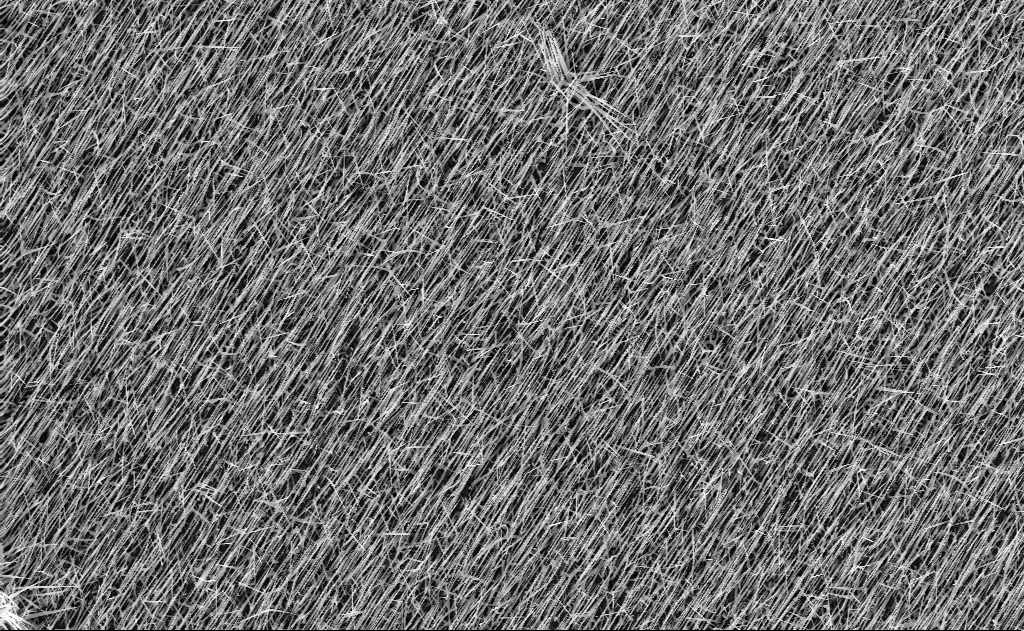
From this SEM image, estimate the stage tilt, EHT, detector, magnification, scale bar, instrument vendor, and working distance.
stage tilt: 0°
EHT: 10 kV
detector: InLens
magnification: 5 K X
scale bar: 10000 nm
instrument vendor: Zeiss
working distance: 7 mm